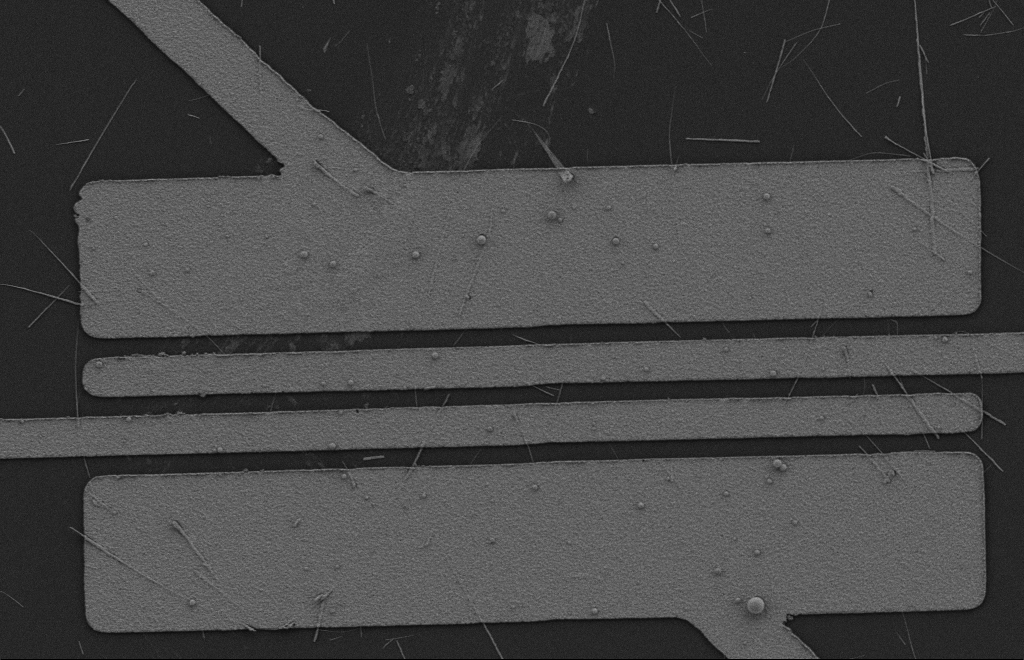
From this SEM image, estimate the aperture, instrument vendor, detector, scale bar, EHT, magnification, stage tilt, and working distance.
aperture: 20 µm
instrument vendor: Zeiss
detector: SE2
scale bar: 2000 nm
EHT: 2 kV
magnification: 5.4 K X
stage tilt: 0°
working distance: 9 mm